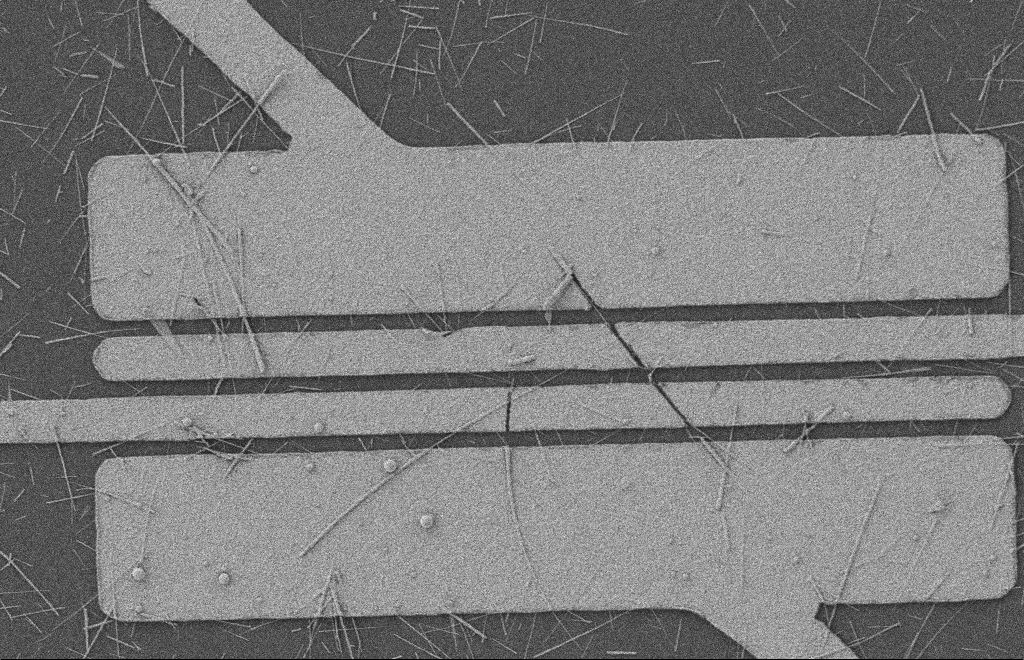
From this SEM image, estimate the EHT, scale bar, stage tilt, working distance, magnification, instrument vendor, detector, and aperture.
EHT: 2 kV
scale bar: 2000 nm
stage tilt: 0°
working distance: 8 mm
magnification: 5.52 K X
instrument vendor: Zeiss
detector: SE2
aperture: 20 µm